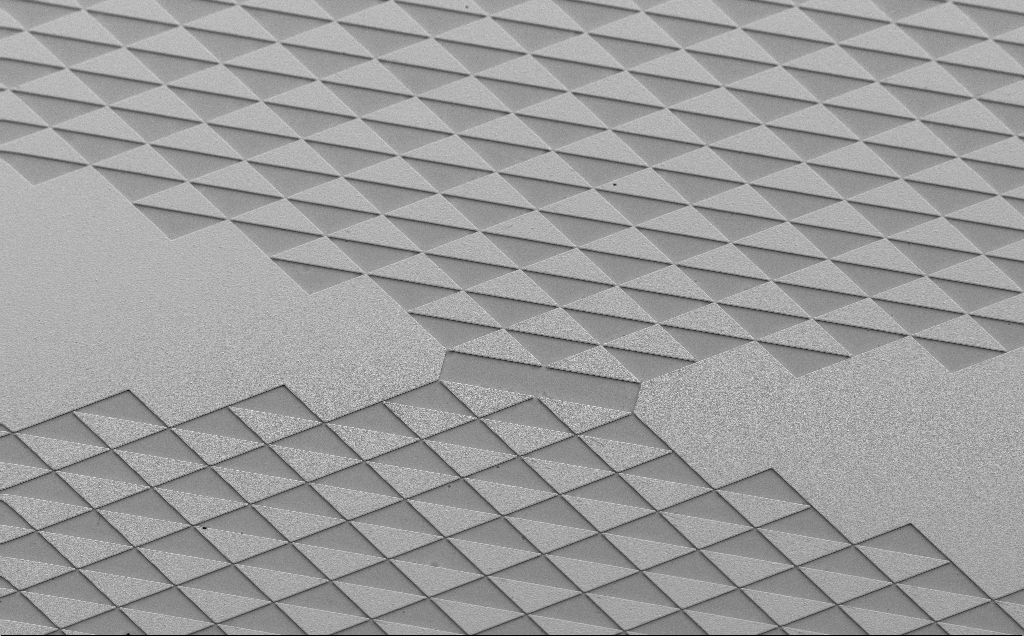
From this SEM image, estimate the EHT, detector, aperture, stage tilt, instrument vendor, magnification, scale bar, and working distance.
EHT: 5 kV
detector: SE2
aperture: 30 µm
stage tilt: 35°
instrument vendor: Zeiss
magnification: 0.389 K X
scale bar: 100000 nm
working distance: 13 mm